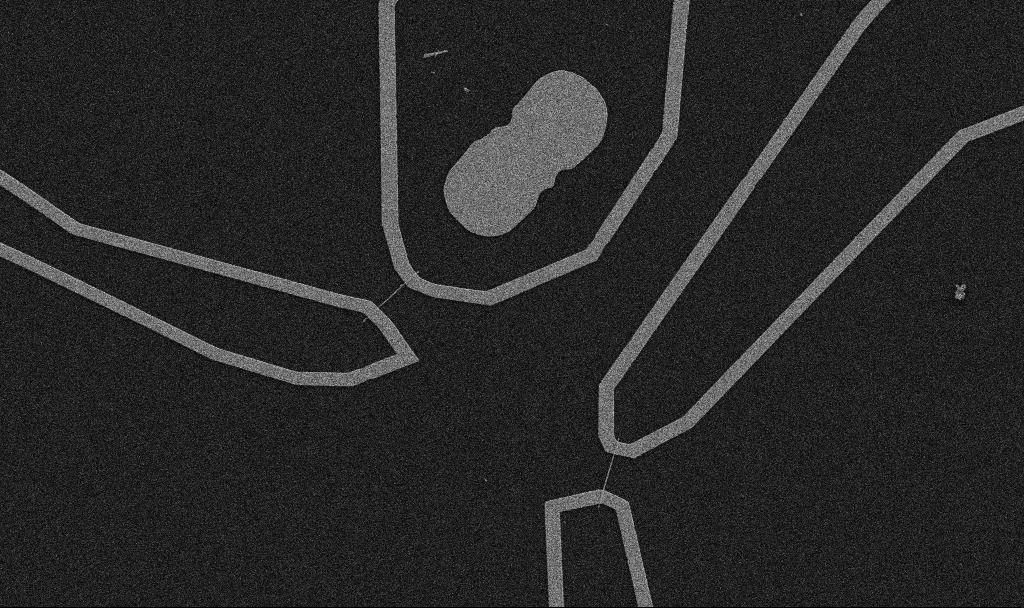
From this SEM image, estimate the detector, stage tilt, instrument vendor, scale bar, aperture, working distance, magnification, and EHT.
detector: SE2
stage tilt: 0°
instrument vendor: Zeiss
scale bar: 10000 nm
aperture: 30 µm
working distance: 10.7 mm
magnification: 5 K X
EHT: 5 kV